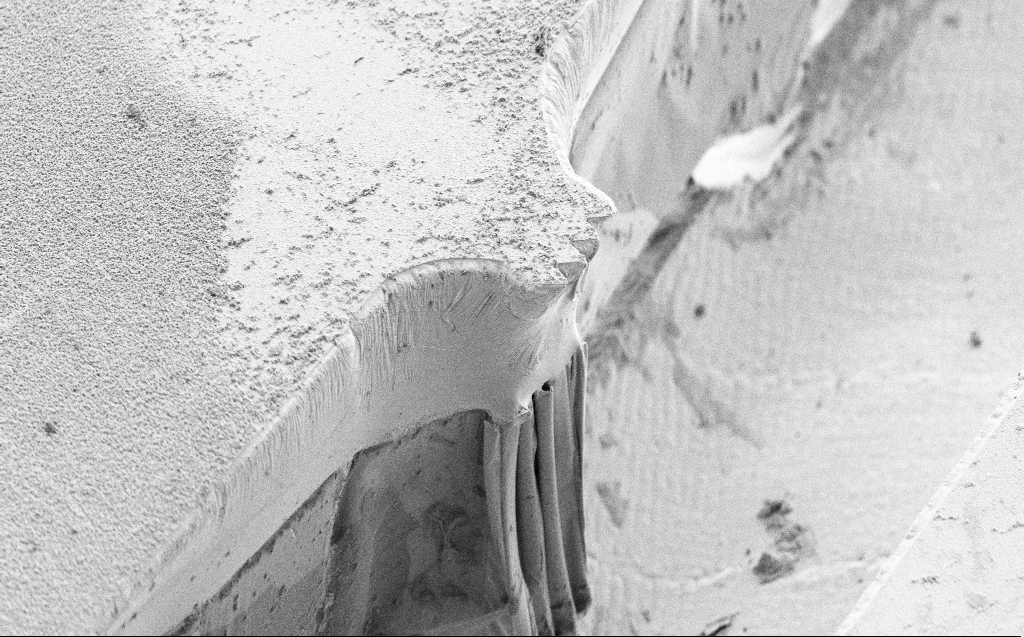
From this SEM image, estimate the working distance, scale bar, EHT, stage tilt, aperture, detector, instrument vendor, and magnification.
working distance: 9 mm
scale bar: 20000 nm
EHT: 5 kV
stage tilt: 45°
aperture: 30 µm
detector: SE2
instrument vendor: Zeiss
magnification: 0.936 K X